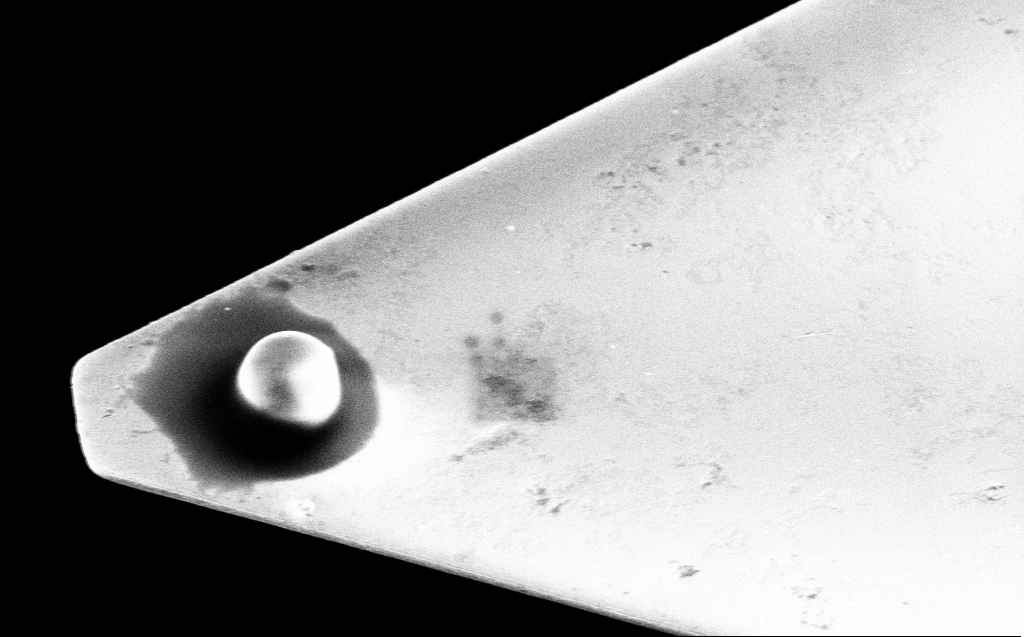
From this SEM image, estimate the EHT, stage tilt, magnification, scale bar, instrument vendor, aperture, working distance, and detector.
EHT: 10 kV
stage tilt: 45°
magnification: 8.33 K X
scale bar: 2000 nm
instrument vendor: Zeiss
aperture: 30 µm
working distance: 12 mm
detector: SE2